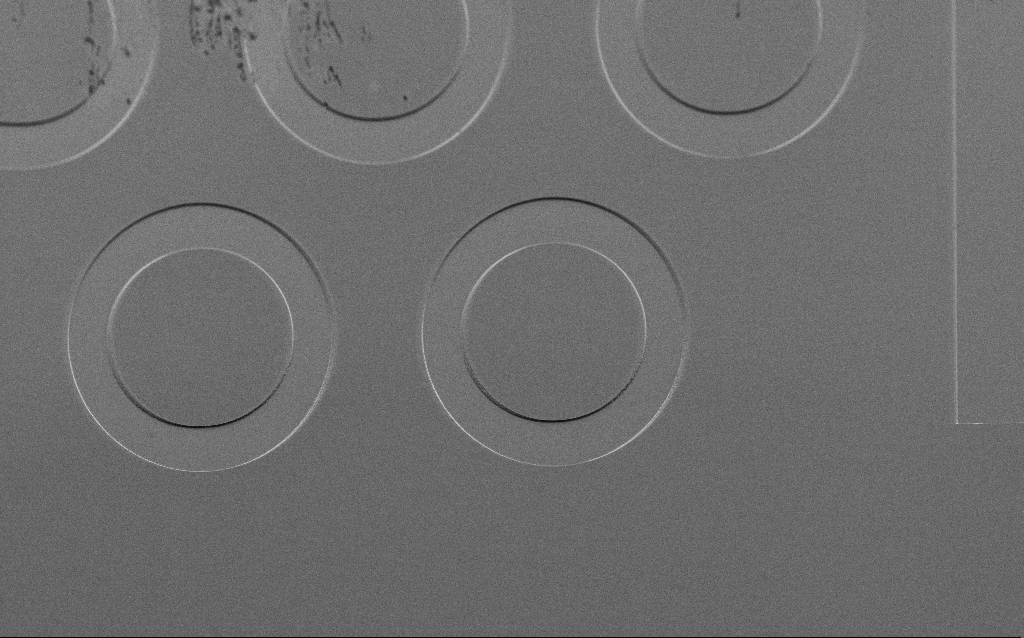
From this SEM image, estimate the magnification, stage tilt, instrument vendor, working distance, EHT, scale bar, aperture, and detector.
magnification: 0.411 K X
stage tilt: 45°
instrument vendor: Zeiss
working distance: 6 mm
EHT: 3 kV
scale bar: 100000 nm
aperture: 30 µm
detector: SE2